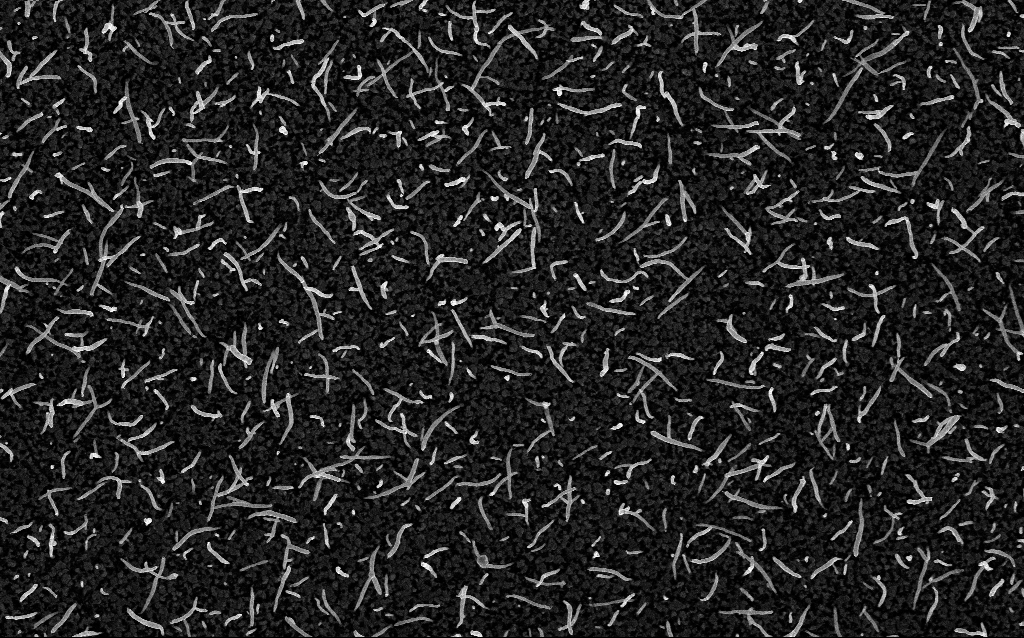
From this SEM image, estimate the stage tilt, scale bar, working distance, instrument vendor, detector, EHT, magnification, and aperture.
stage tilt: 0°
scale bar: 1000 nm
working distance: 2.6 mm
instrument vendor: Zeiss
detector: InLens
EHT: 5 kV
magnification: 20 K X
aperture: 30 µm